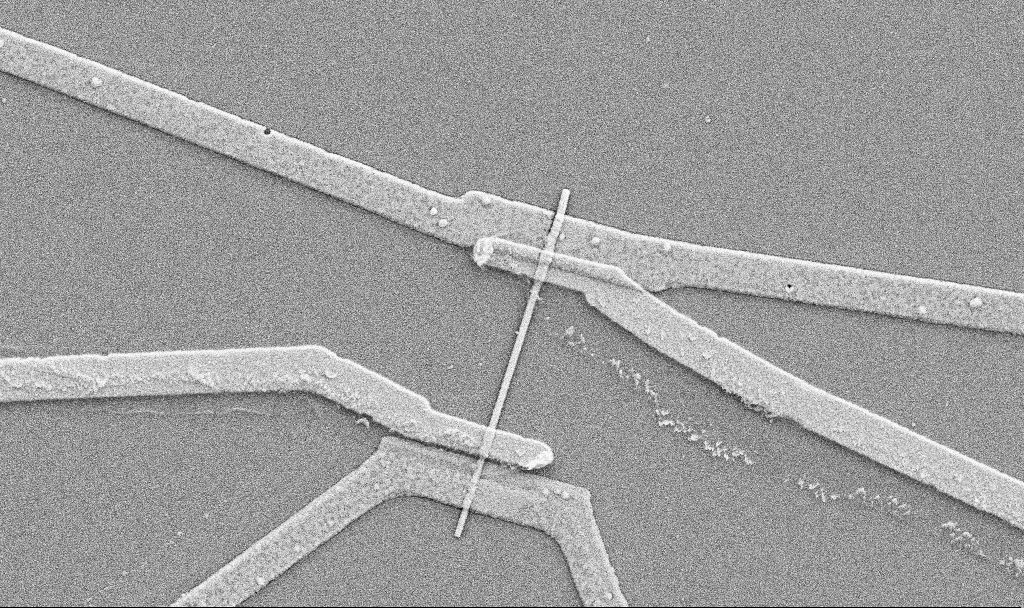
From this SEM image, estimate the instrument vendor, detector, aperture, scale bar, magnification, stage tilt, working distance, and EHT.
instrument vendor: Zeiss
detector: SE2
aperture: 30 µm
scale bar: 1000 nm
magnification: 20 K X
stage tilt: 0°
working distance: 10.7 mm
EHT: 5 kV